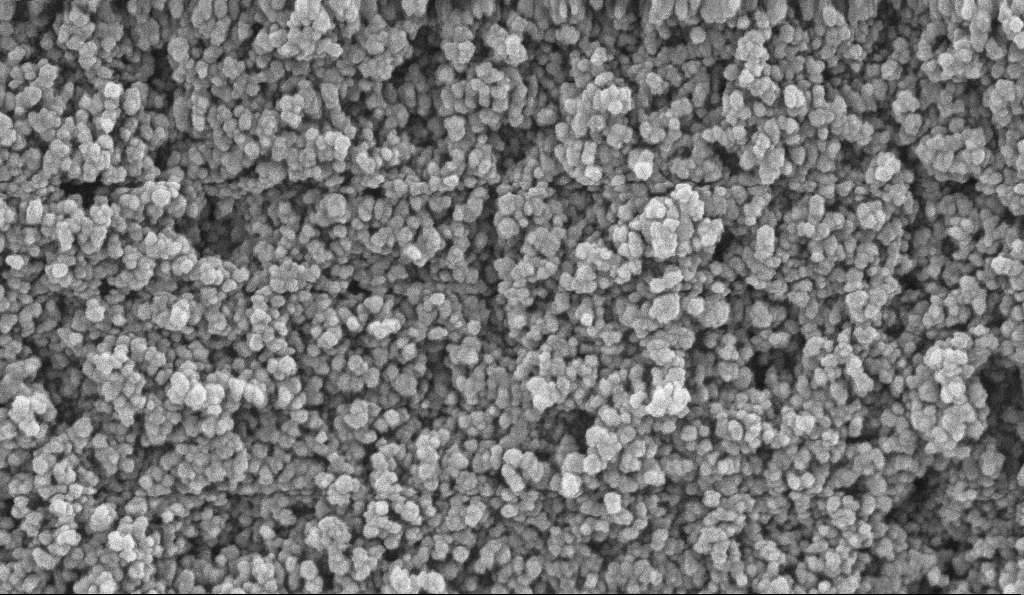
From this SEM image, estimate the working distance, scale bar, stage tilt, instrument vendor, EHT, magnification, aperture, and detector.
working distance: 5.1 mm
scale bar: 200 nm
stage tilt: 0°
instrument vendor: Zeiss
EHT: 10 kV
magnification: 135 K X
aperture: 30 µm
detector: InLens